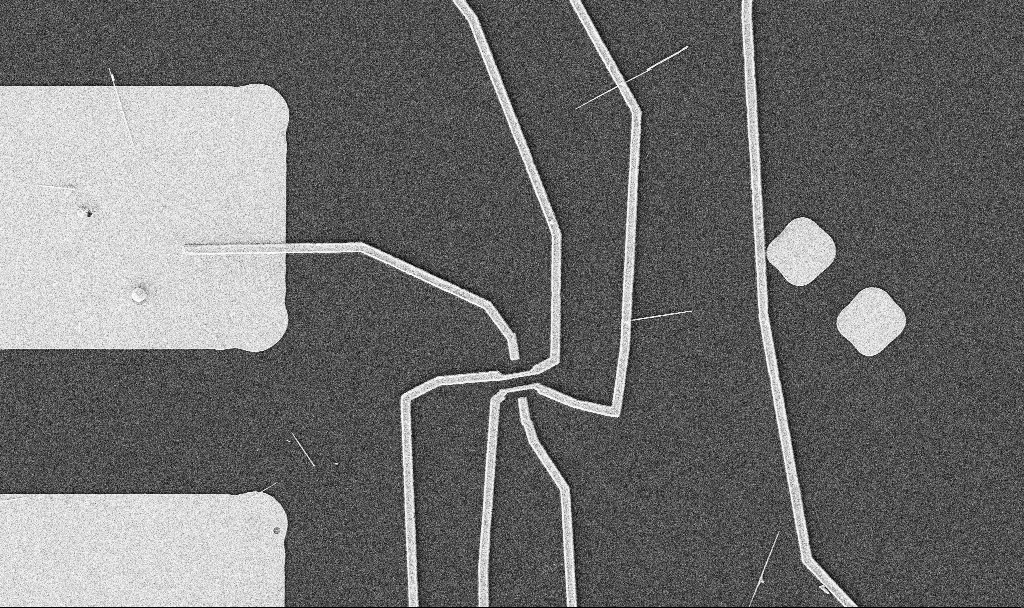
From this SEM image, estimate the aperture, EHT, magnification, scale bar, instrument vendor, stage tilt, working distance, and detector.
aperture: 30 µm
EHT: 5 kV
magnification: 5 K X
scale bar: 10000 nm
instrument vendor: Zeiss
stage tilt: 0°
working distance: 10.7 mm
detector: SE2